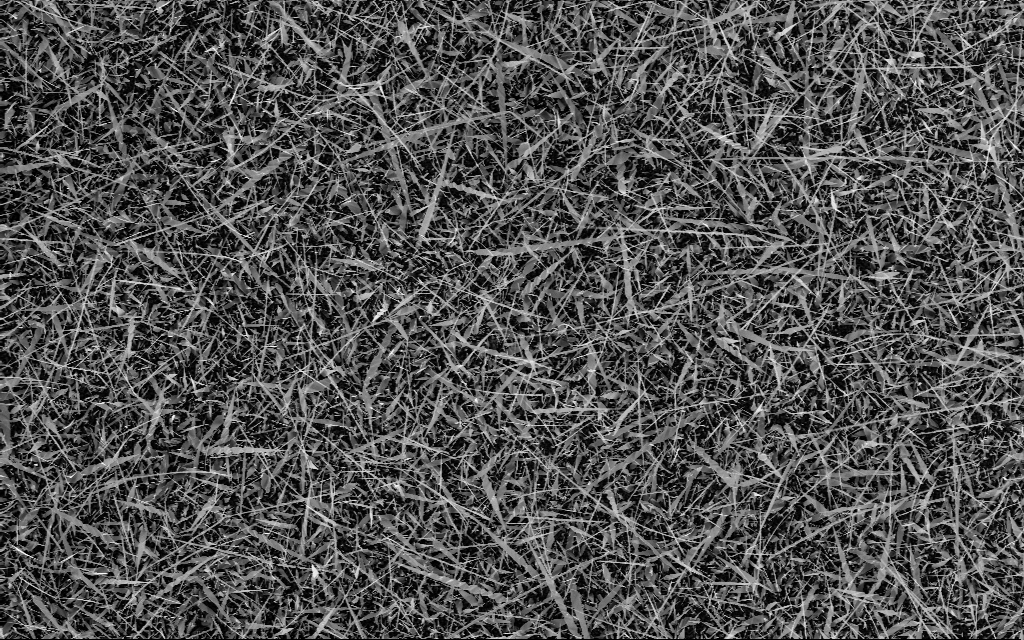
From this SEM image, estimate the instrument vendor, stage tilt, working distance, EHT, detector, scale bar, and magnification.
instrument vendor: Zeiss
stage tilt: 0°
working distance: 6 mm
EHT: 10 kV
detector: InLens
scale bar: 10000 nm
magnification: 3 K X